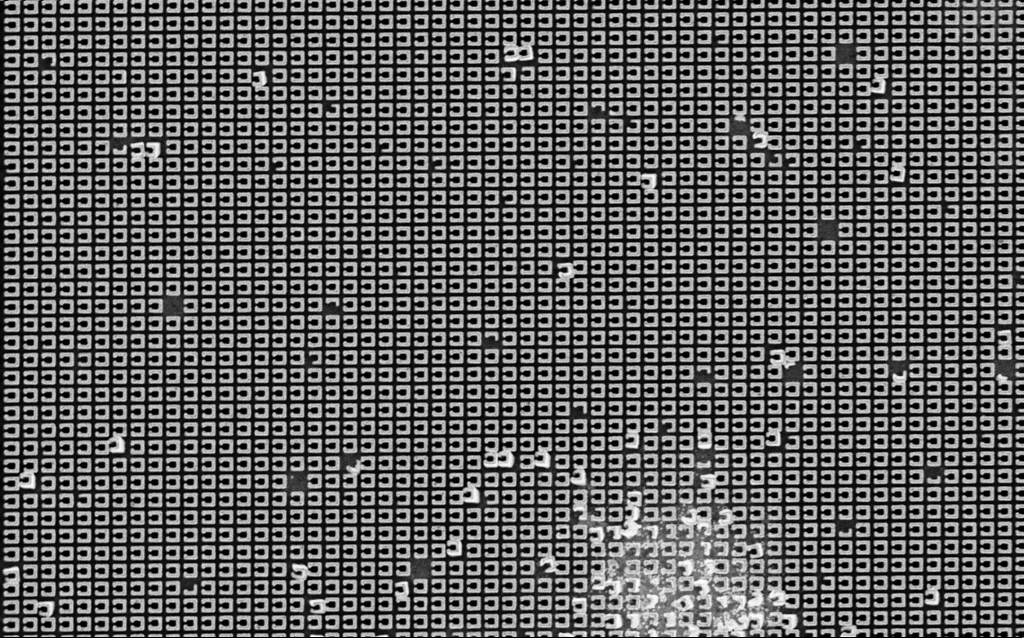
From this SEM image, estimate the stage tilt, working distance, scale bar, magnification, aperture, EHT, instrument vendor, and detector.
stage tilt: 0°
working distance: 5 mm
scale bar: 2000 nm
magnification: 14.13 K X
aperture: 30 µm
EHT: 3 kV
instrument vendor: Zeiss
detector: InLens